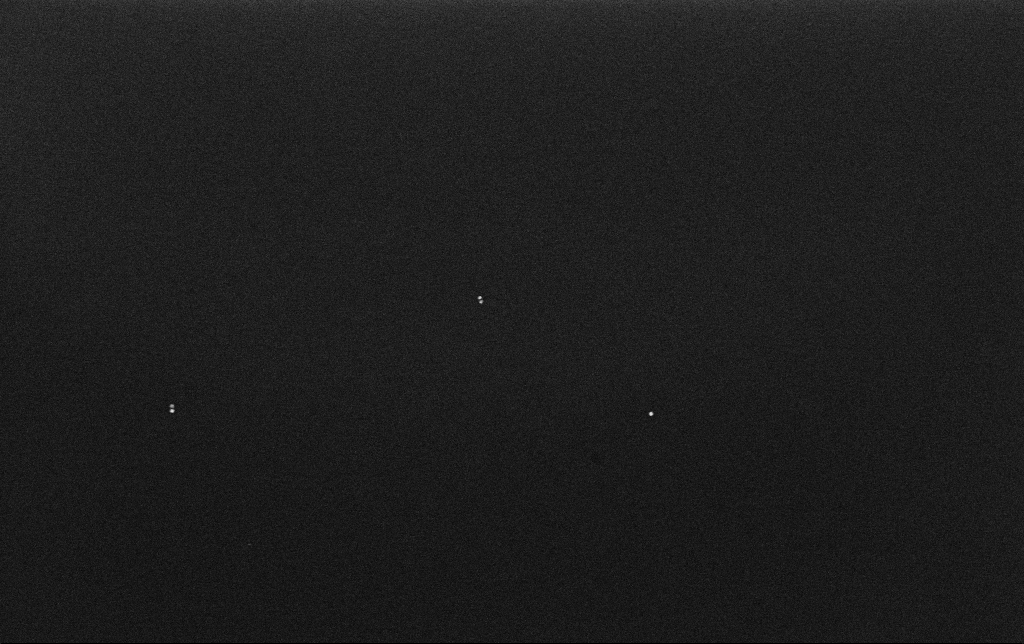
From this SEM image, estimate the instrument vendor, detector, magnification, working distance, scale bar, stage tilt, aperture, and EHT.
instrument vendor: Zeiss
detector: InLens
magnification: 100 K X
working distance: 3.2 mm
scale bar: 200 nm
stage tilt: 0°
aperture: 30 µm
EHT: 10 kV